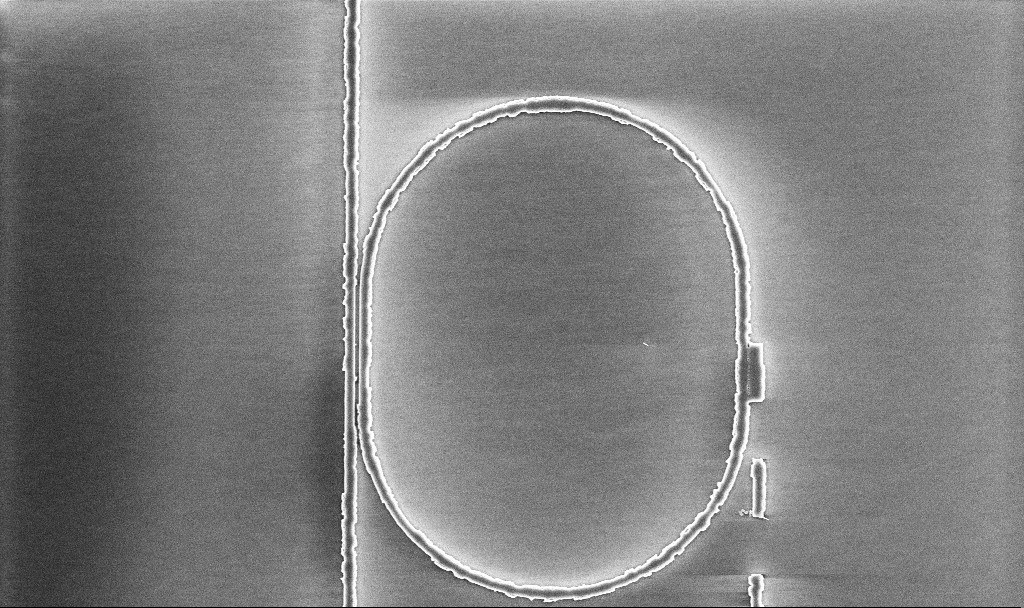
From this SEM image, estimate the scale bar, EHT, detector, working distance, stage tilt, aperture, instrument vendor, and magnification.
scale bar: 10000 nm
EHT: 5 kV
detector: InLens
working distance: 10.1 mm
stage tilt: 0°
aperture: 30 µm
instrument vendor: Zeiss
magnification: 7.18 K X